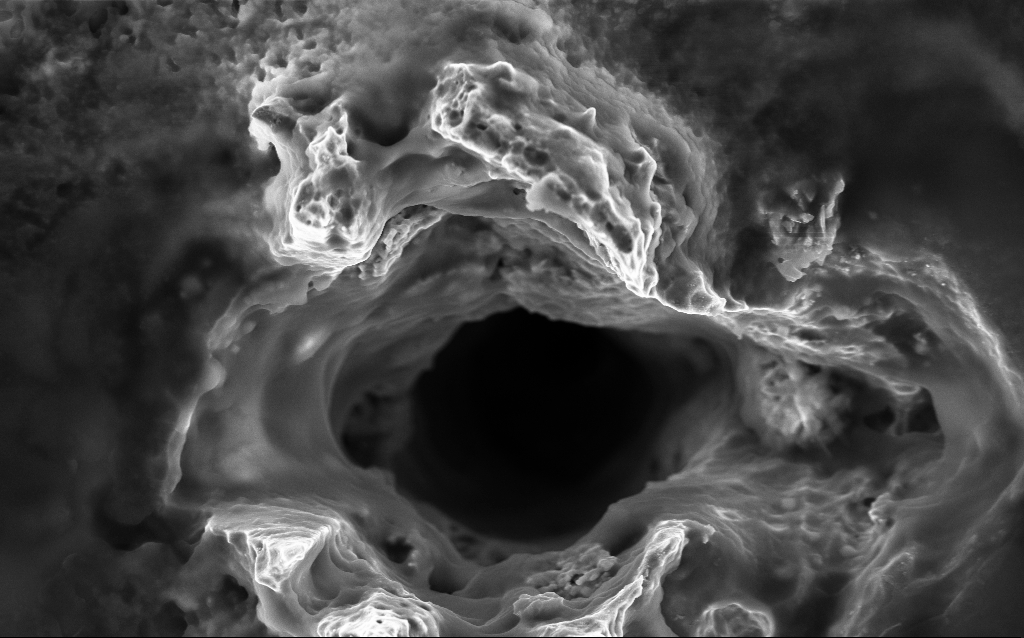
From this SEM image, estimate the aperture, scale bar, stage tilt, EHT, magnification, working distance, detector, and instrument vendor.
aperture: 30 µm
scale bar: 2000 nm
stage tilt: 0°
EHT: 5 kV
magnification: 20.35 K X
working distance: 4.6 mm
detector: InLens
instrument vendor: Zeiss